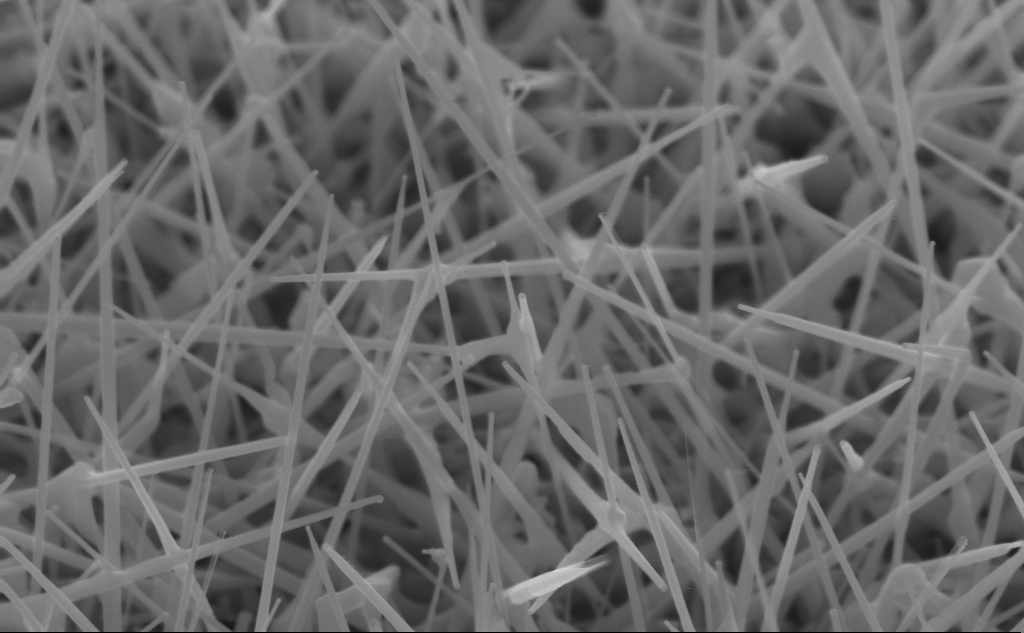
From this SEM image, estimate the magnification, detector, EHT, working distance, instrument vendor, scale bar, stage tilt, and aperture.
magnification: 67.97 K X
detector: InLens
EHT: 10 kV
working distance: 4 mm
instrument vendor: Zeiss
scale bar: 1000 nm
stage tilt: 45°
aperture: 30 µm